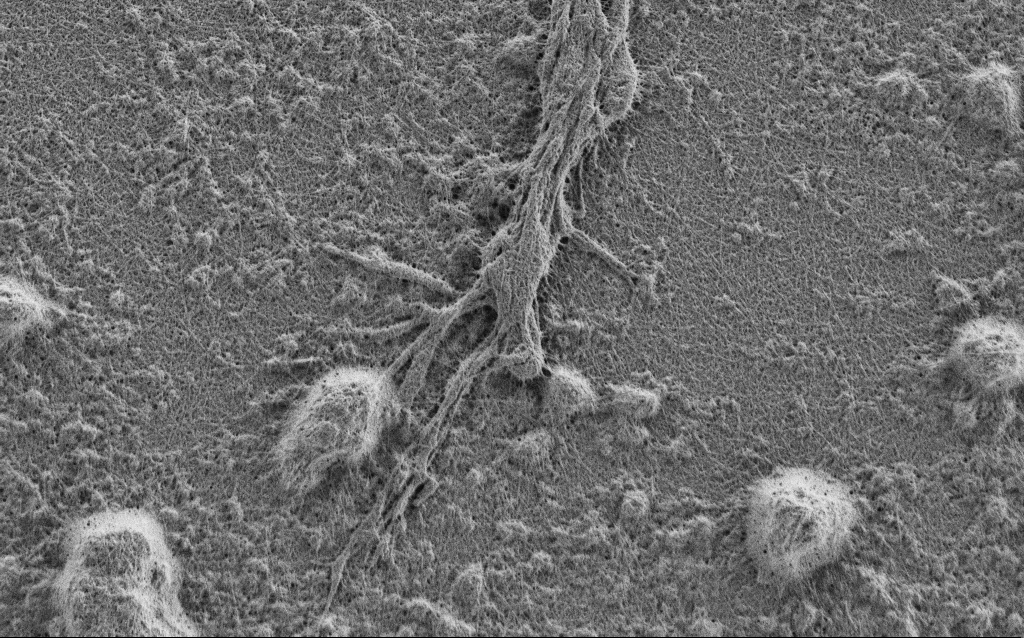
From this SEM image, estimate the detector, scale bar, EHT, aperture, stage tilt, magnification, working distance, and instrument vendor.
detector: SE2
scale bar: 2000 nm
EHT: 0.9 kV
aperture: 30 µm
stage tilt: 0°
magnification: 10 K X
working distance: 4 mm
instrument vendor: Zeiss